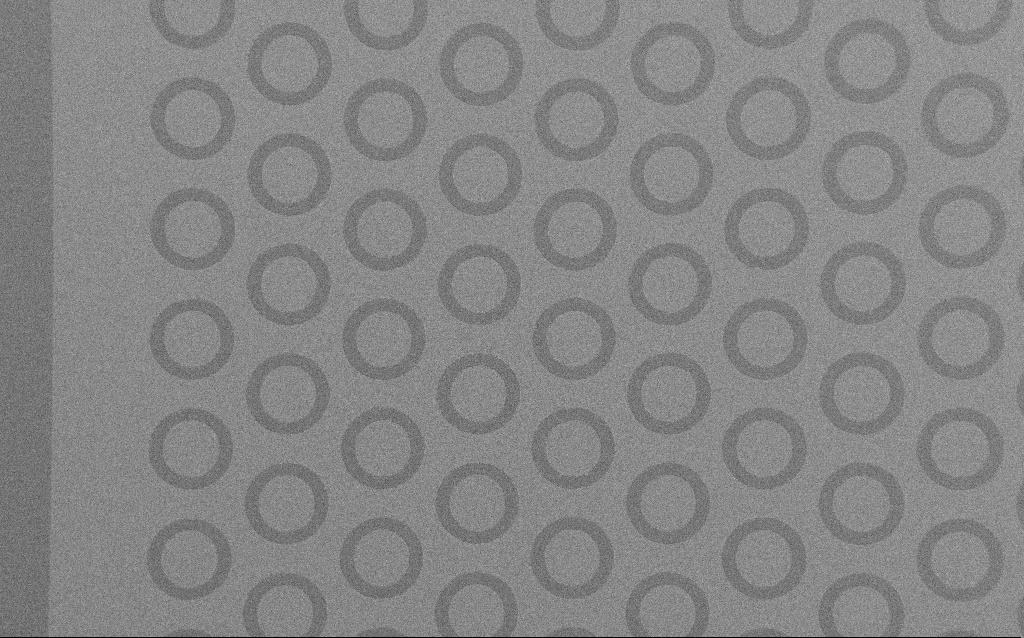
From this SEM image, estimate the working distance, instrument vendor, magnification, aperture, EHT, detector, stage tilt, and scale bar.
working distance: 5 mm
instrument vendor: Zeiss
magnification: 0.255 K X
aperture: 30 µm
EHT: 2 kV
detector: SE2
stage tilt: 0°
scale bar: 100000 nm